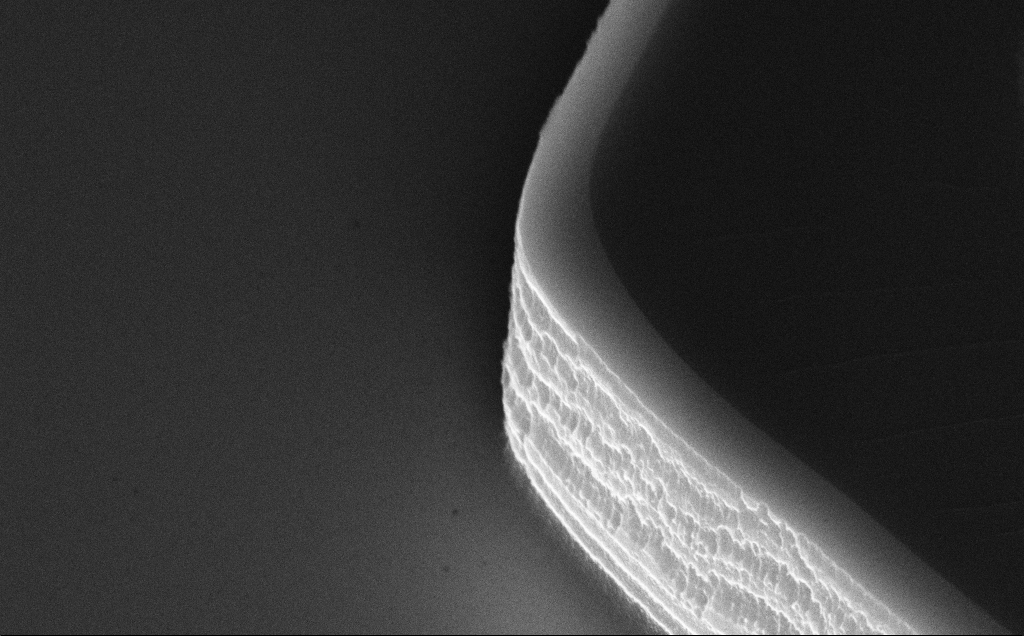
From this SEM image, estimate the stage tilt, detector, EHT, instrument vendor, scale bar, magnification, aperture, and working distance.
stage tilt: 50°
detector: InLens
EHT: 10 kV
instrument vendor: Zeiss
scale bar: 2000 nm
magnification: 34.5 K X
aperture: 30 µm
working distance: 10 mm